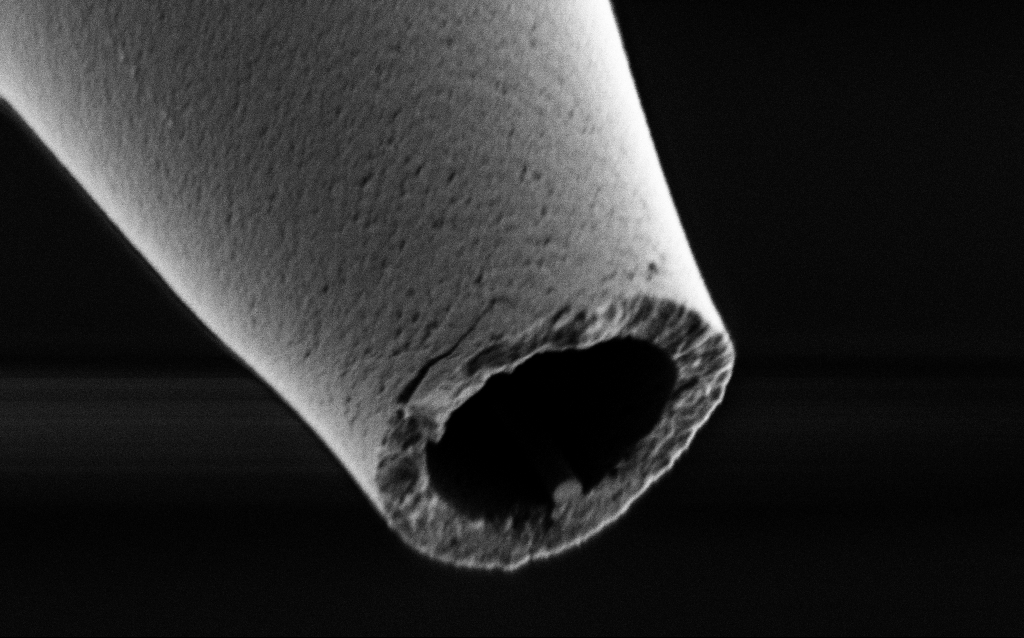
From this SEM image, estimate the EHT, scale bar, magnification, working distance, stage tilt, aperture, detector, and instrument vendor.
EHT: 1 kV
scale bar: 1000 nm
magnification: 50 K X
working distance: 7 mm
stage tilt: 45°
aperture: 30 µm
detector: SE2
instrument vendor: Zeiss